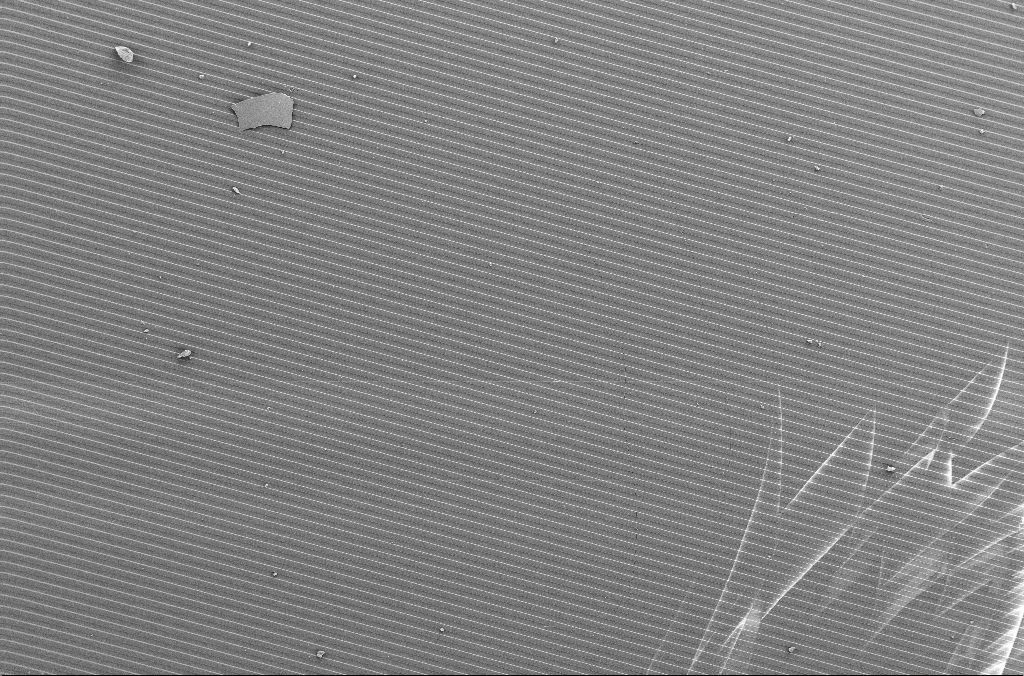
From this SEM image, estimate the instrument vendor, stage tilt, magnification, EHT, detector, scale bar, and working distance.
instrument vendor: Zeiss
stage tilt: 0°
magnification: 0.2 K X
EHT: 5 kV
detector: SE2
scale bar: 100000 nm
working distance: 4.1 mm